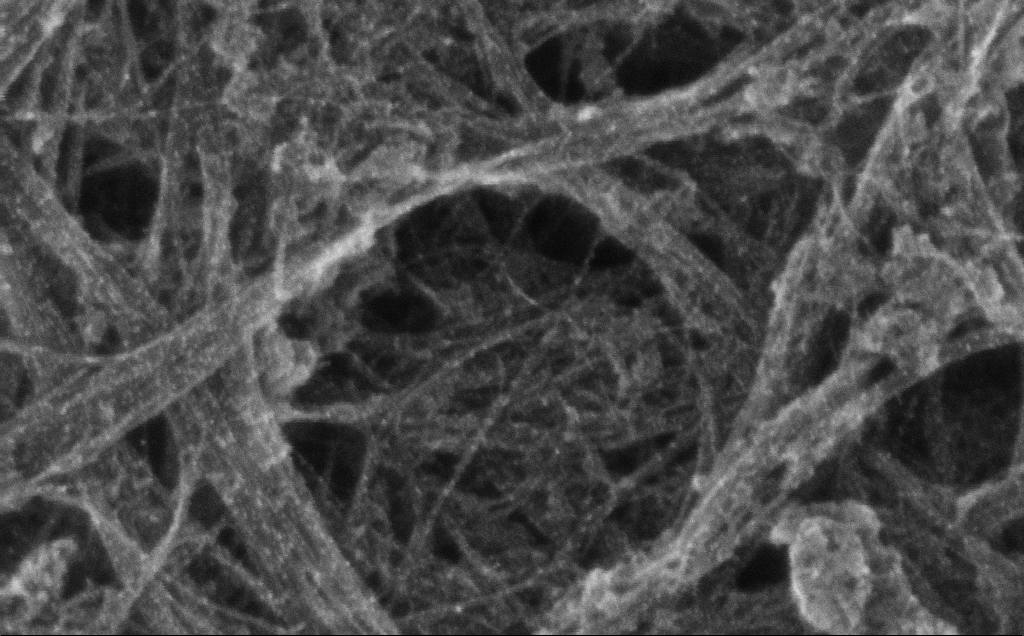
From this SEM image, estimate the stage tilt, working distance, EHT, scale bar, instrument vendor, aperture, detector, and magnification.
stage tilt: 0°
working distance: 3 mm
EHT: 10 kV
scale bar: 200 nm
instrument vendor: Zeiss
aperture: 30 µm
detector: InLens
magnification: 241.5 K X